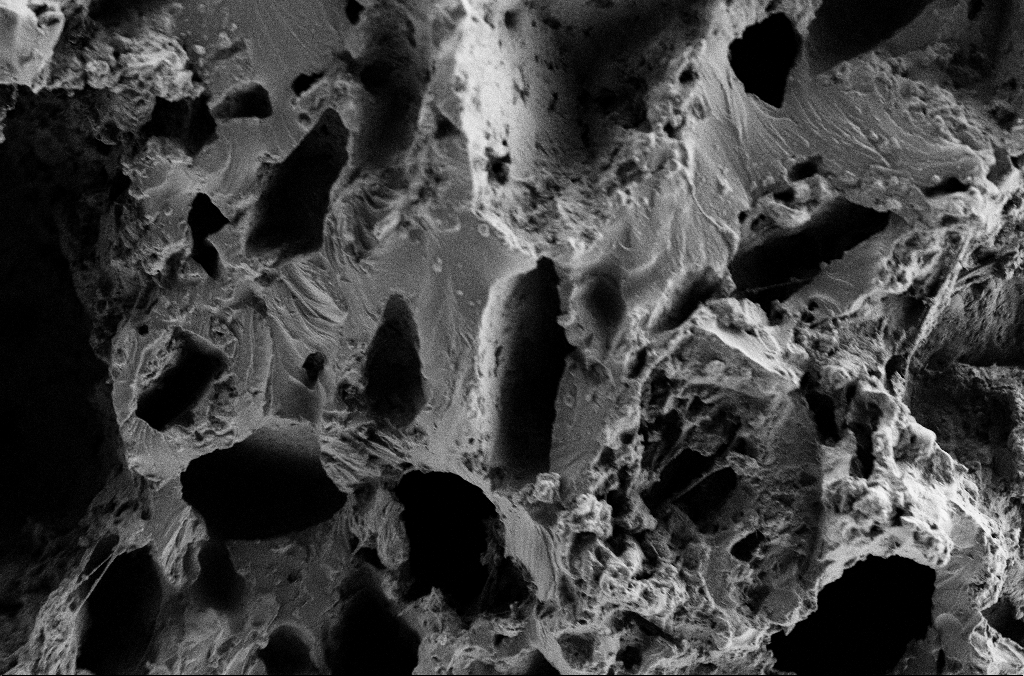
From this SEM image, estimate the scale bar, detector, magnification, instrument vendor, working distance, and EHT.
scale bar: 20000 nm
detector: SE2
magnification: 1 K X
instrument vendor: Zeiss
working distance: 3 mm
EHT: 2 kV